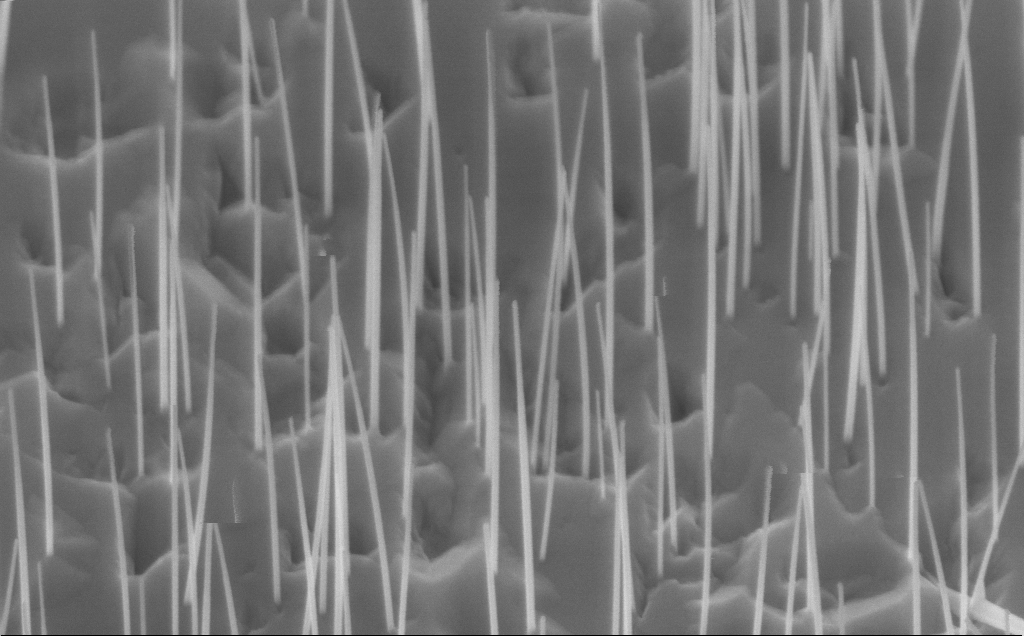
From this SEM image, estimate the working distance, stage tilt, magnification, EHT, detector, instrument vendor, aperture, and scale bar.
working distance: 7 mm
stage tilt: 45°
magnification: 80 K X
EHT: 10 kV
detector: InLens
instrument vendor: Zeiss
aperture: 30 µm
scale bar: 200 nm